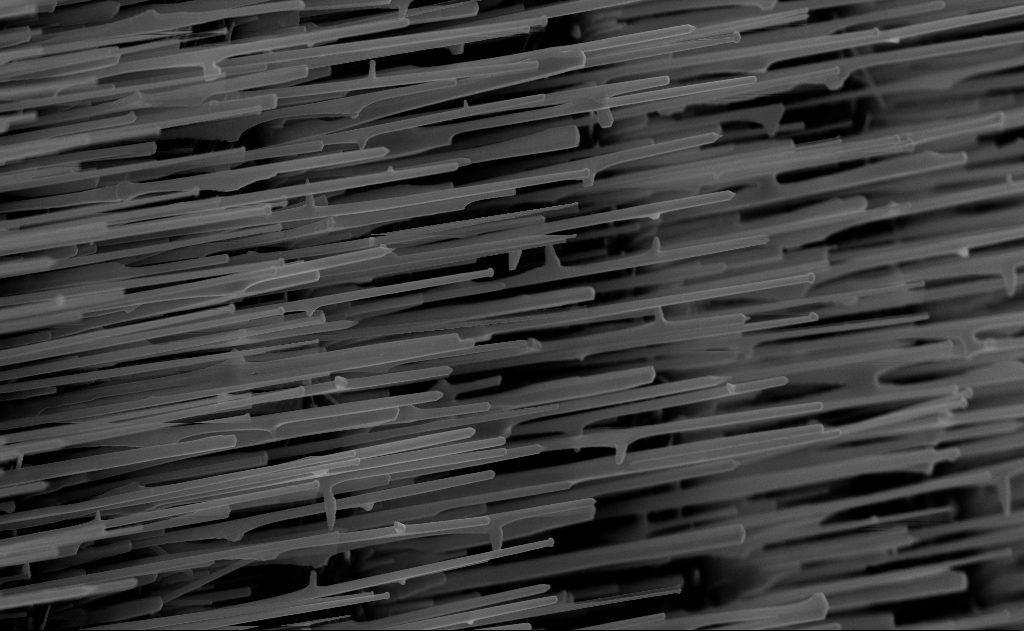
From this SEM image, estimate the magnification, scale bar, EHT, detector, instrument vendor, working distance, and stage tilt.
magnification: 20 K X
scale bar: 2000 nm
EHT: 10 kV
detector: InLens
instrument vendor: Zeiss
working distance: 7 mm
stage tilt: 0°